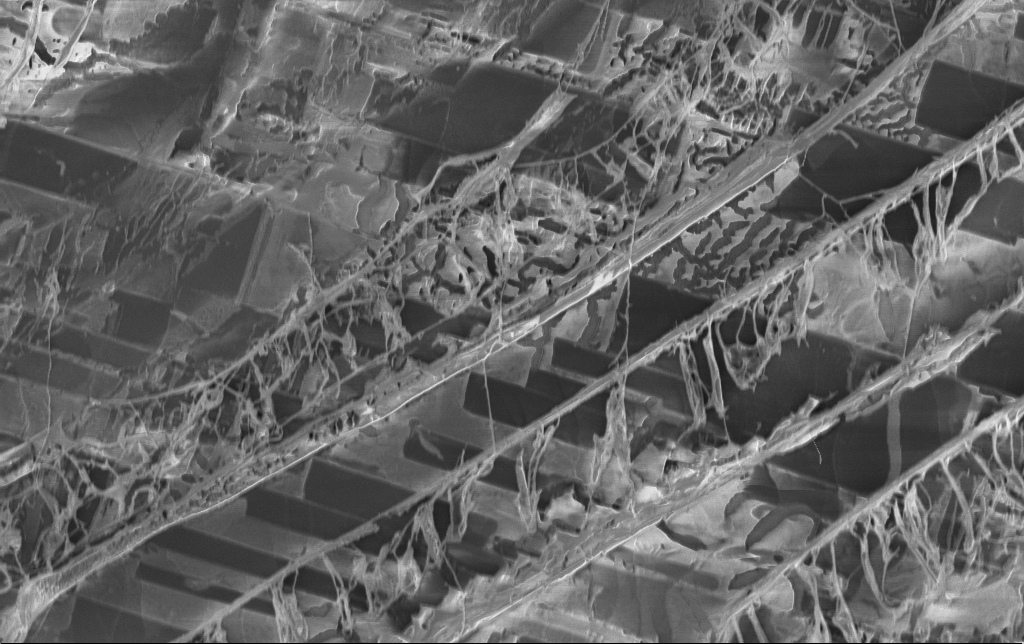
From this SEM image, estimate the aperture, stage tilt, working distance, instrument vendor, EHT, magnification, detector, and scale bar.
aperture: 30 µm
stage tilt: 0°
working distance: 3.1 mm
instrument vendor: Zeiss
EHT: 1.5 kV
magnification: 4.35 K X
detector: InLens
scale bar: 10000 nm